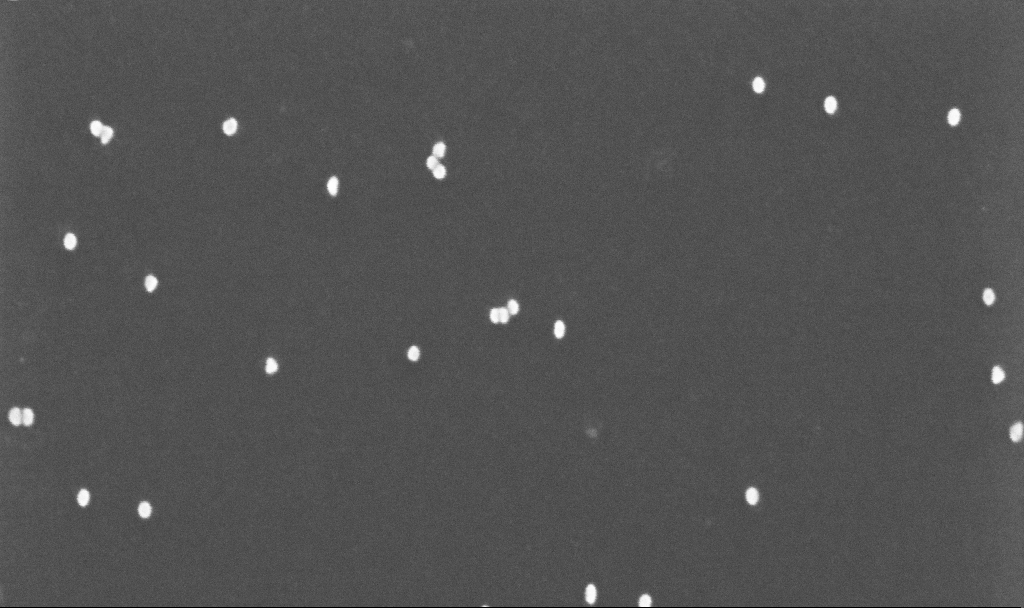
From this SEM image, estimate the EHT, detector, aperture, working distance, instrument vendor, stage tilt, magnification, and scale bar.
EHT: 10 kV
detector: InLens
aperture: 30 µm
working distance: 3.3 mm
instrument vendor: Zeiss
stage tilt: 0°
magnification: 200 K X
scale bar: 200 nm